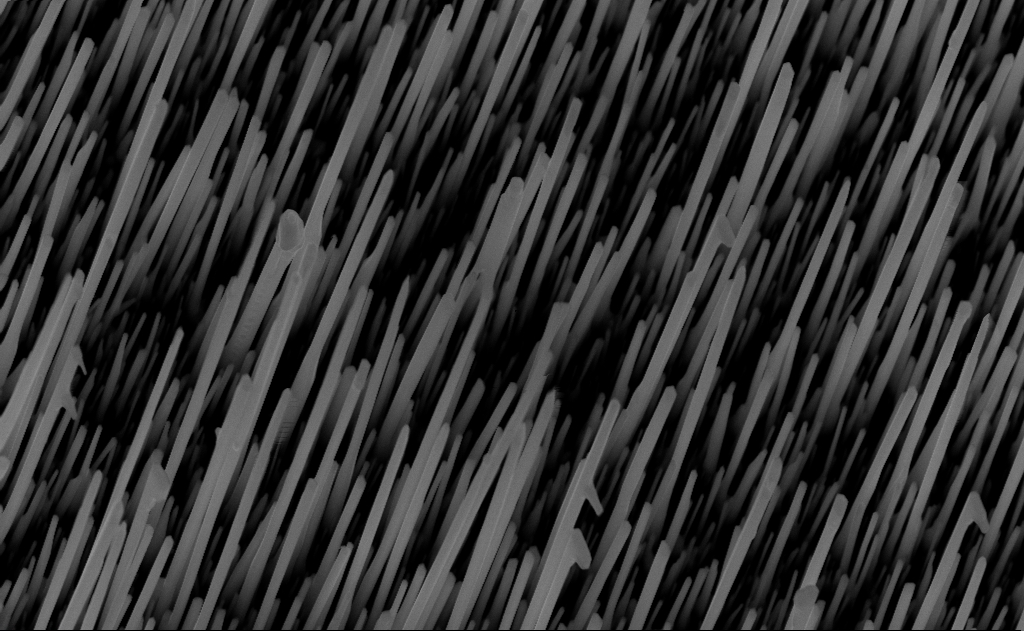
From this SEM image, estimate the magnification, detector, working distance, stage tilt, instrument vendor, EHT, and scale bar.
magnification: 40 K X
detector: InLens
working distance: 7 mm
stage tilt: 0°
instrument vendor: Zeiss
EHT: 10 kV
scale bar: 1000 nm